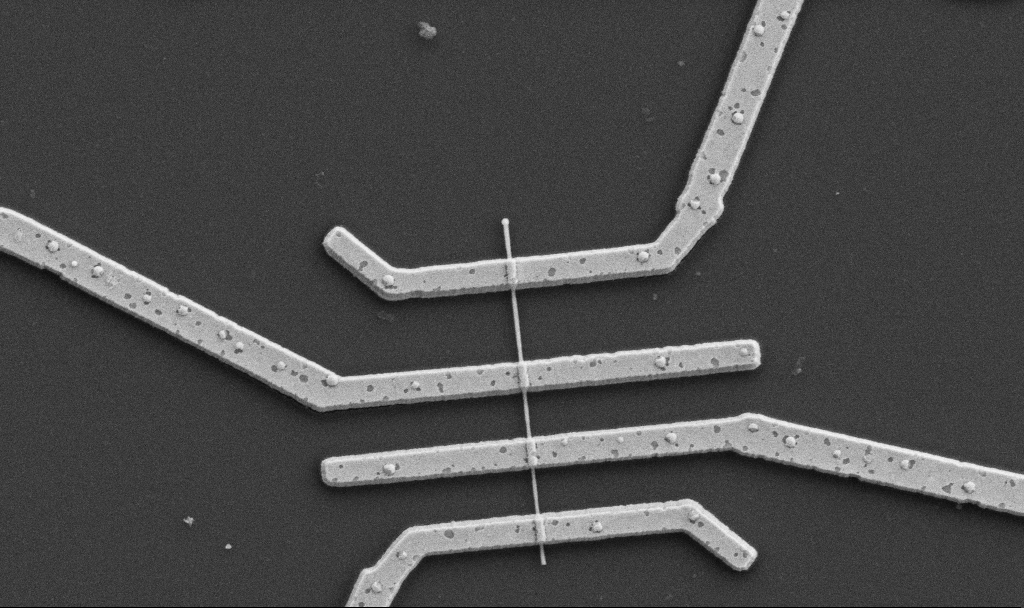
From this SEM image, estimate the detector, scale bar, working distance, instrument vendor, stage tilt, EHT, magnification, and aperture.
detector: SE2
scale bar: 1000 nm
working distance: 10.6 mm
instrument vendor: Zeiss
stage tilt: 0°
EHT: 5 kV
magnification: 20 K X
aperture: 30 µm